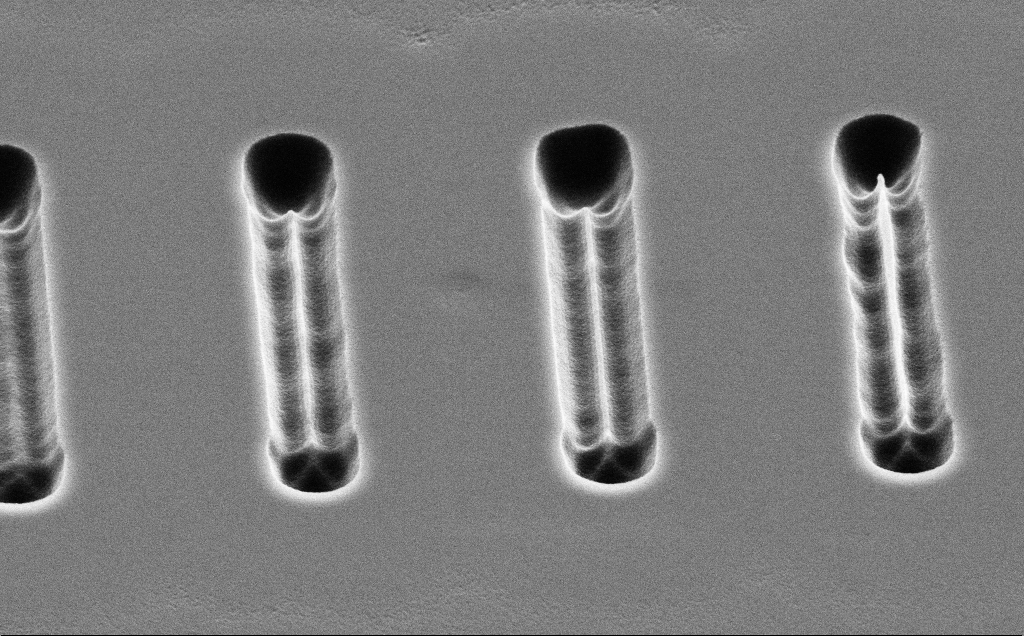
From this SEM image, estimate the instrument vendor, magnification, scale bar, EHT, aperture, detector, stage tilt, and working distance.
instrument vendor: Zeiss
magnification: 16.67 K X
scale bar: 2000 nm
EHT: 5 kV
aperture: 30 µm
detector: SE2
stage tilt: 45°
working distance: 10 mm